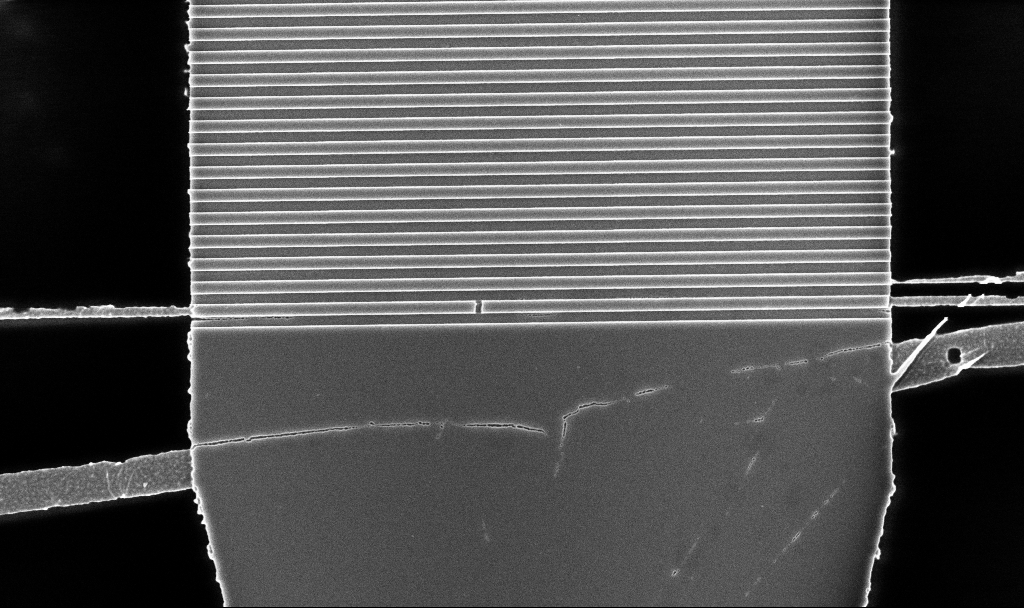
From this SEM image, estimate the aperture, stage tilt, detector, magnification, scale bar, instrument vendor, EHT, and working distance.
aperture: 30 µm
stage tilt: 0°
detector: InLens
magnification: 13.03 K X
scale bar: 2000 nm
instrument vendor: Zeiss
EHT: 5 kV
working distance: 5.2 mm